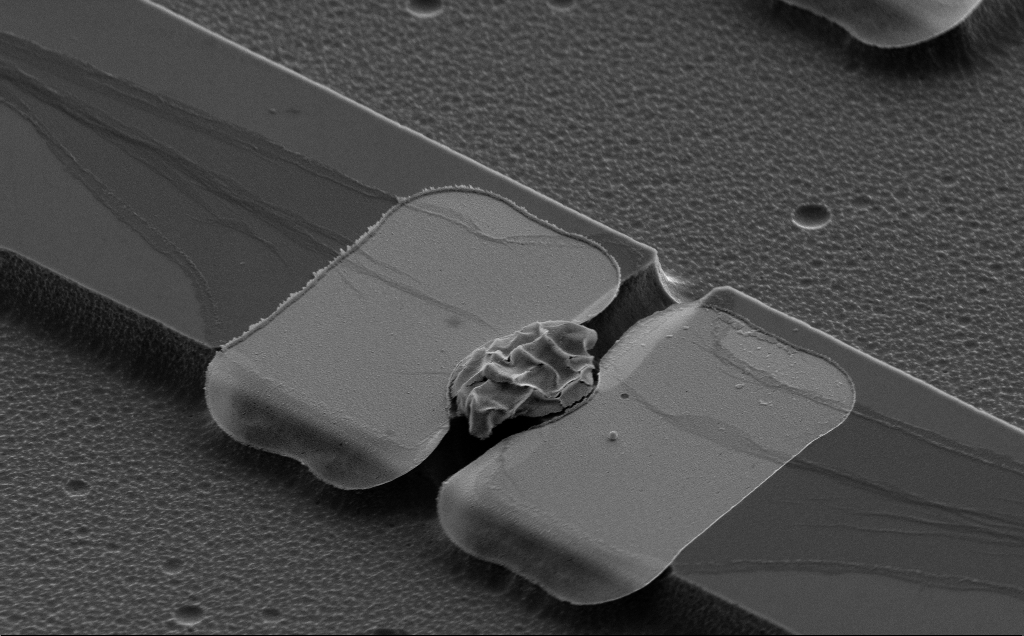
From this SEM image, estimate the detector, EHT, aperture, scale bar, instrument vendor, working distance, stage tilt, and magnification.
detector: SE2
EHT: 5 kV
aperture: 30 µm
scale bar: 2000 nm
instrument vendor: Zeiss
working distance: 10 mm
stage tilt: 50°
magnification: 8.08 K X